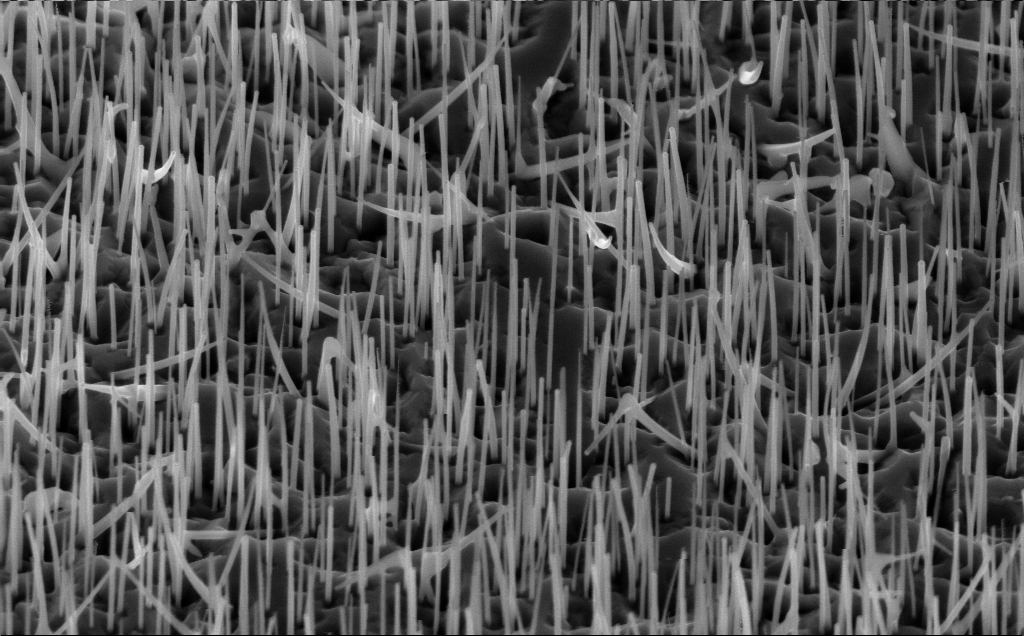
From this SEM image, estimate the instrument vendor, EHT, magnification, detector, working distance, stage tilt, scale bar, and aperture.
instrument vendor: Zeiss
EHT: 10 kV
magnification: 40 K X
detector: InLens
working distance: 5 mm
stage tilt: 45°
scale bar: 1000 nm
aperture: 30 µm